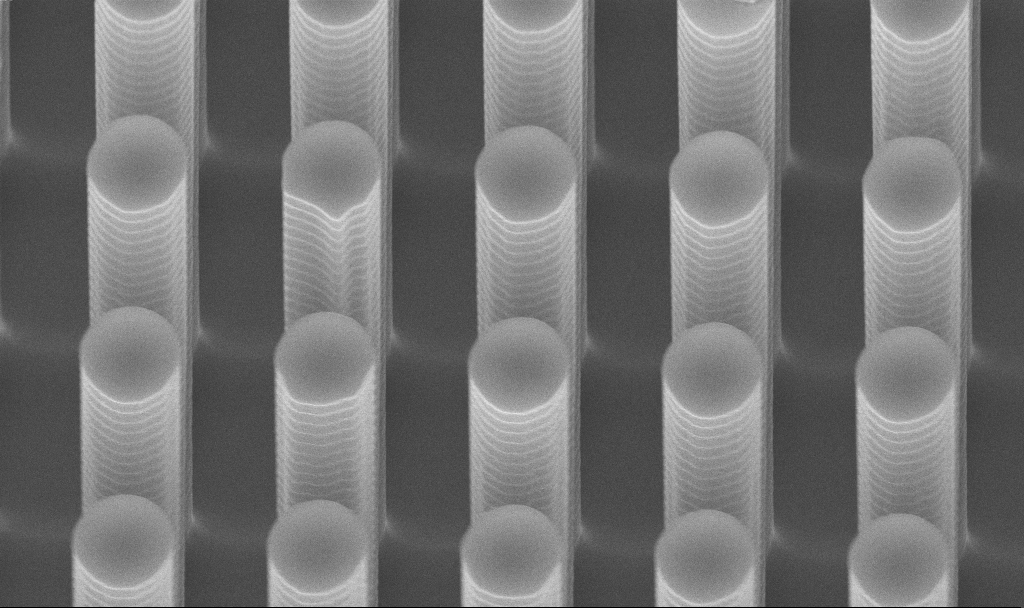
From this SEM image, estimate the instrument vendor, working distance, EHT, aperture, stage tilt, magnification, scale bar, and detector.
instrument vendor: Zeiss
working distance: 8.2 mm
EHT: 10 kV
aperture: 30 µm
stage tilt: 45°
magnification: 18 K X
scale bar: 2000 nm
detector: InLens